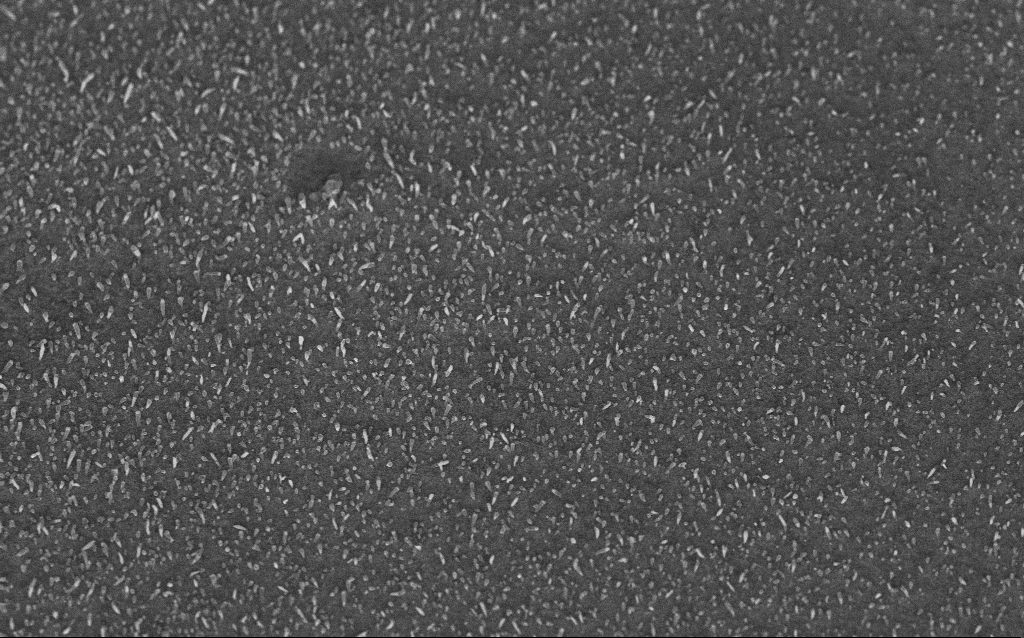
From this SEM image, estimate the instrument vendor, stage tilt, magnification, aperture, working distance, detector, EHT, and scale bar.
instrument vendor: Zeiss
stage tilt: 45°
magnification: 20 K X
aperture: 30 µm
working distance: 7.2 mm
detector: InLens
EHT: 5 kV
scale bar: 1000 nm